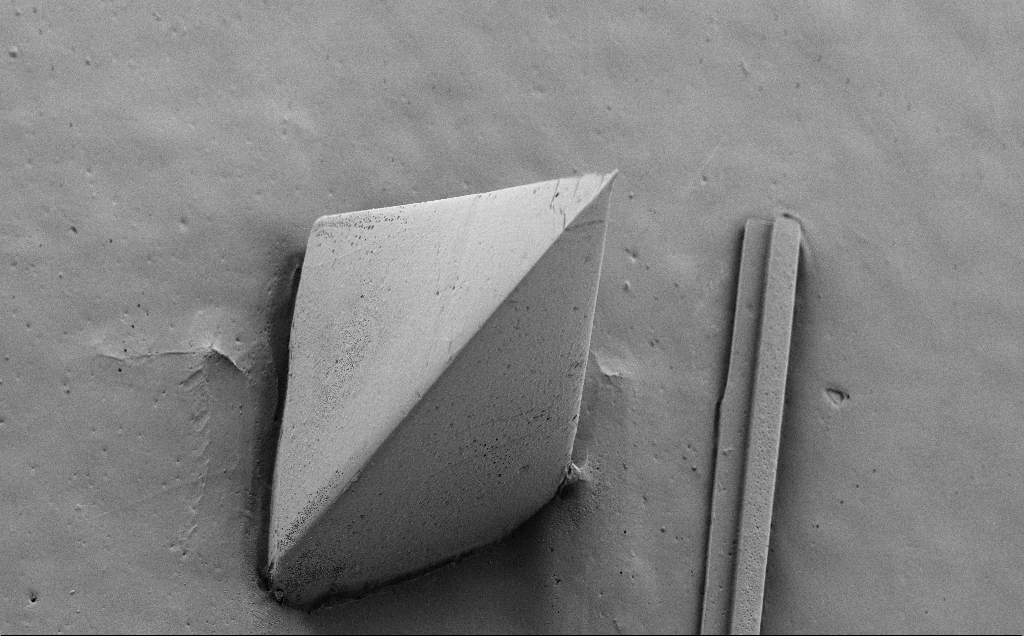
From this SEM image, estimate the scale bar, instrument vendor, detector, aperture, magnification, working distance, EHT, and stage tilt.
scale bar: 100000 nm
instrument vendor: Zeiss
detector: SE2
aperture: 30 µm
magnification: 0.196 K X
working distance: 8 mm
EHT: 5 kV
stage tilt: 40°